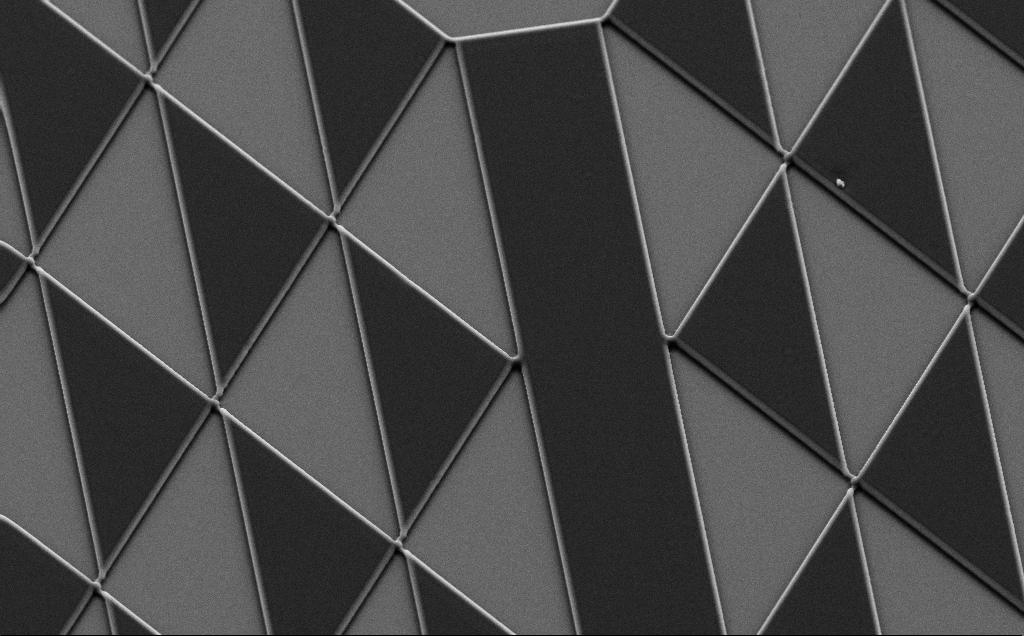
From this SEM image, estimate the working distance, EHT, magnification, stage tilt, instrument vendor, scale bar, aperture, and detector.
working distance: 8 mm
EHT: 10 kV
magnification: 1.59 K X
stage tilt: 35°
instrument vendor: Zeiss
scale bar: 20000 nm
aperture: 30 µm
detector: SE2